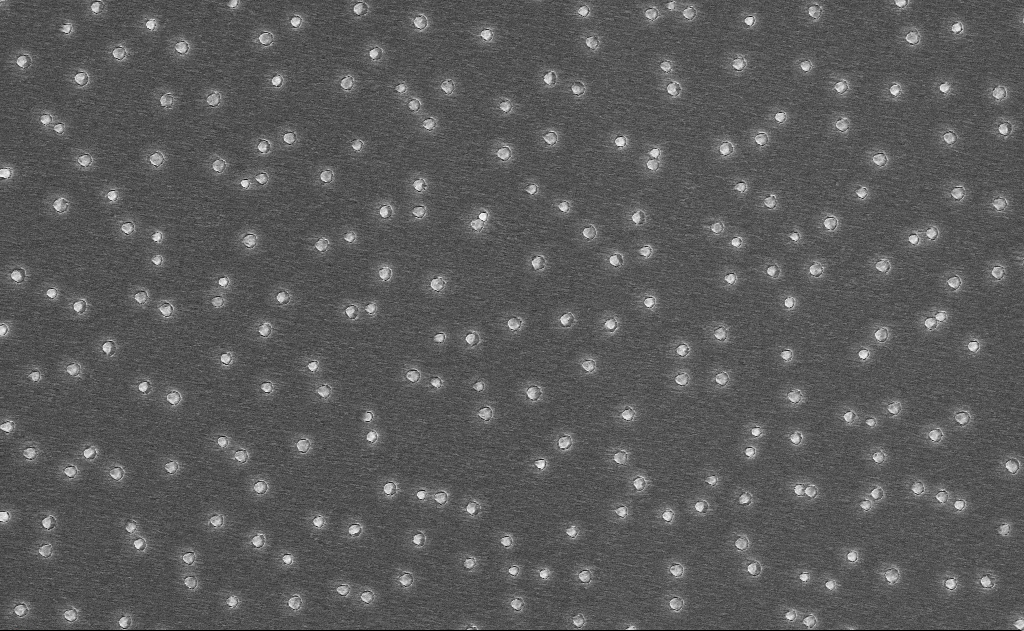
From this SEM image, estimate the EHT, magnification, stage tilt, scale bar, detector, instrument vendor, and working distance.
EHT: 10 kV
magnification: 10 K X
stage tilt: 0°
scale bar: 2000 nm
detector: InLens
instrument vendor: Zeiss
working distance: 16 mm